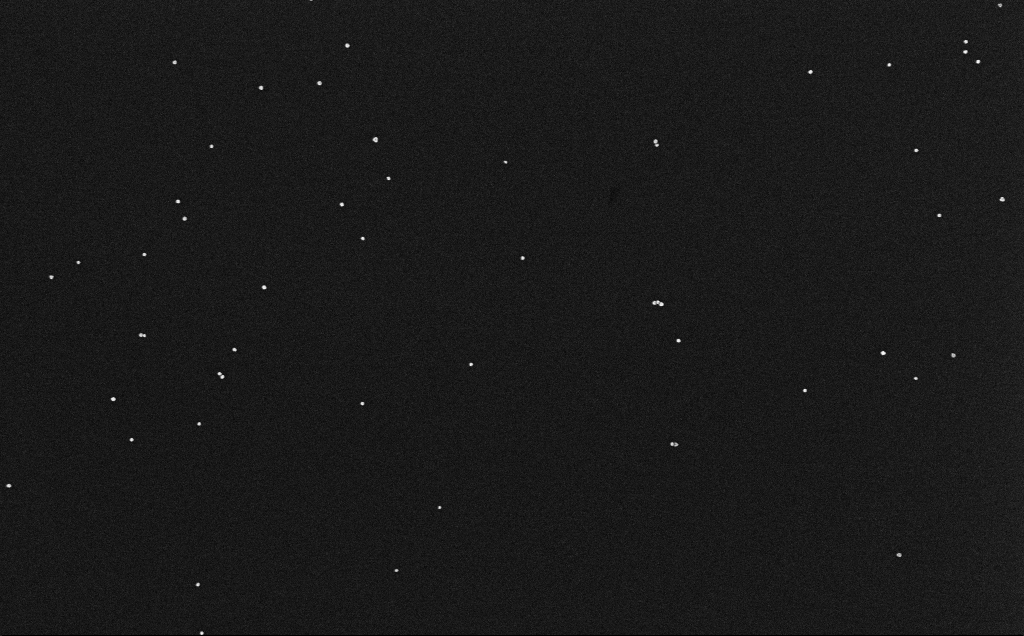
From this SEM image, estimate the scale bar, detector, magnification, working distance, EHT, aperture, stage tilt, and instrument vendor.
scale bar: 200 nm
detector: InLens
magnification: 100 K X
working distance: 3.2 mm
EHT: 10 kV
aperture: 30 µm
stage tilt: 0°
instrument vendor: Zeiss